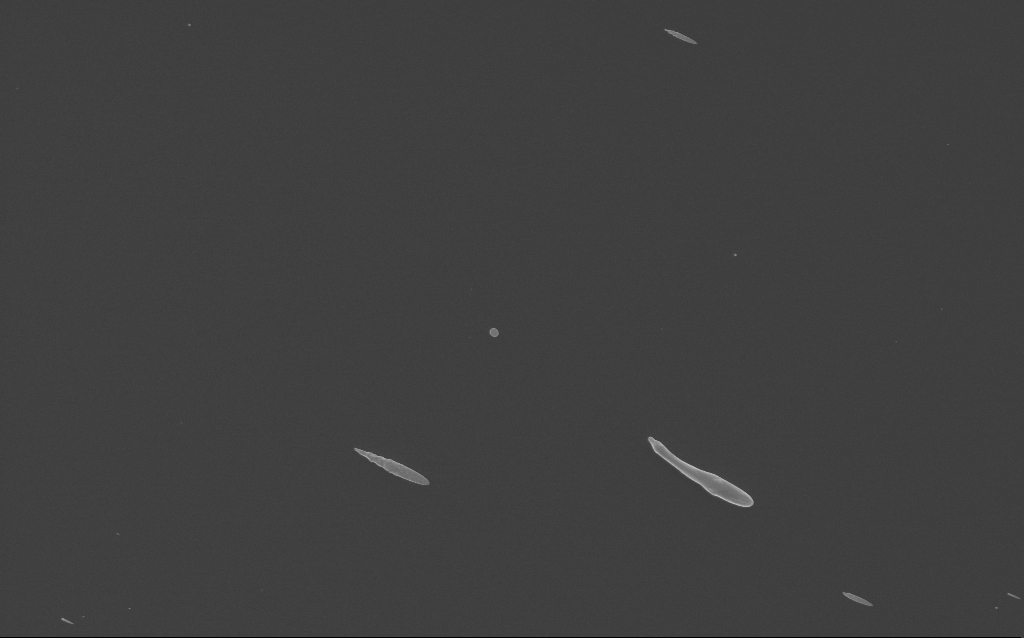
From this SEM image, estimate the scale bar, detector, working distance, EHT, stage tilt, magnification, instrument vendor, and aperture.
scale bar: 2000 nm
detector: InLens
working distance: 3 mm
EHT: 3 kV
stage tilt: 0°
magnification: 7.3 K X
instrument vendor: Zeiss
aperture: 30 µm